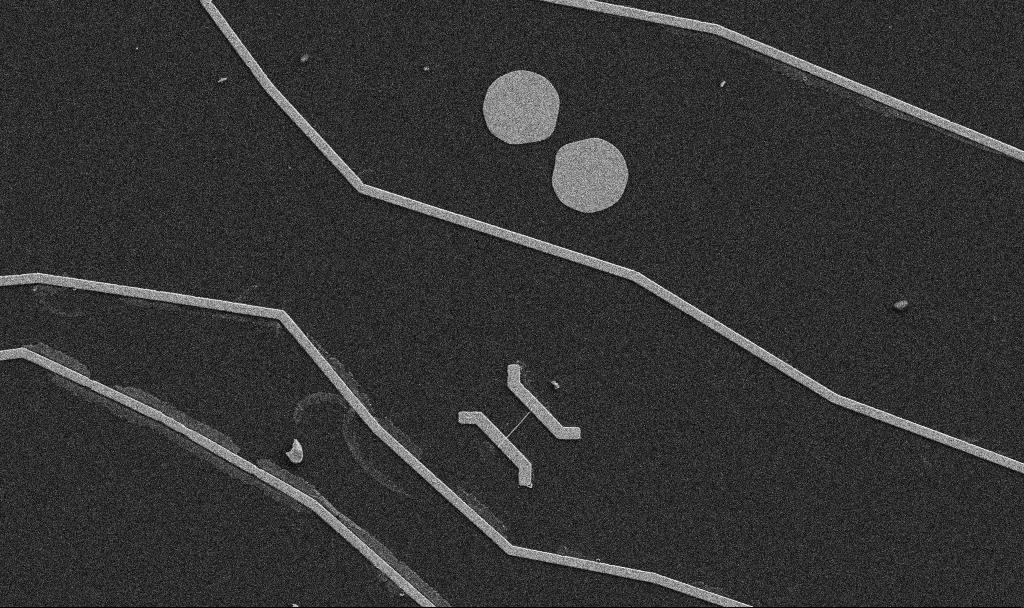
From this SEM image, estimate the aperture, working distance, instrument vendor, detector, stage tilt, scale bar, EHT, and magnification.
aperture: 30 µm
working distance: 10.7 mm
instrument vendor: Zeiss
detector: SE2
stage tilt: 0°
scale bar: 10000 nm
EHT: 5 kV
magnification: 5 K X